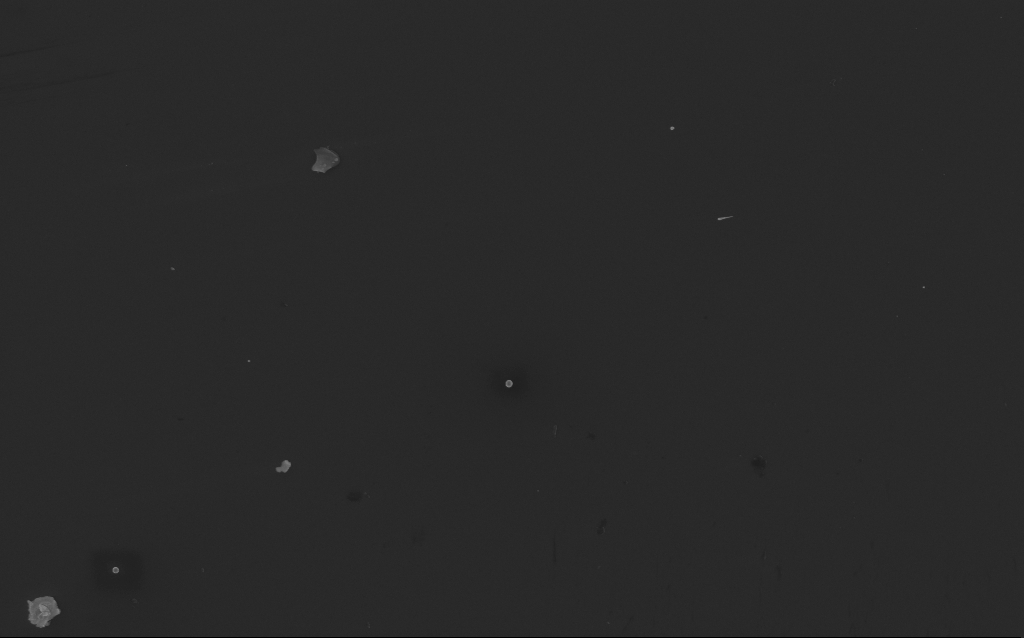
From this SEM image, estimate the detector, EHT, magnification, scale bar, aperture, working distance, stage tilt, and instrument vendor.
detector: InLens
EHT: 5 kV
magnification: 12.07 K X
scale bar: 1000 nm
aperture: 30 µm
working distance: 3 mm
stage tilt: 0°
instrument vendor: Zeiss